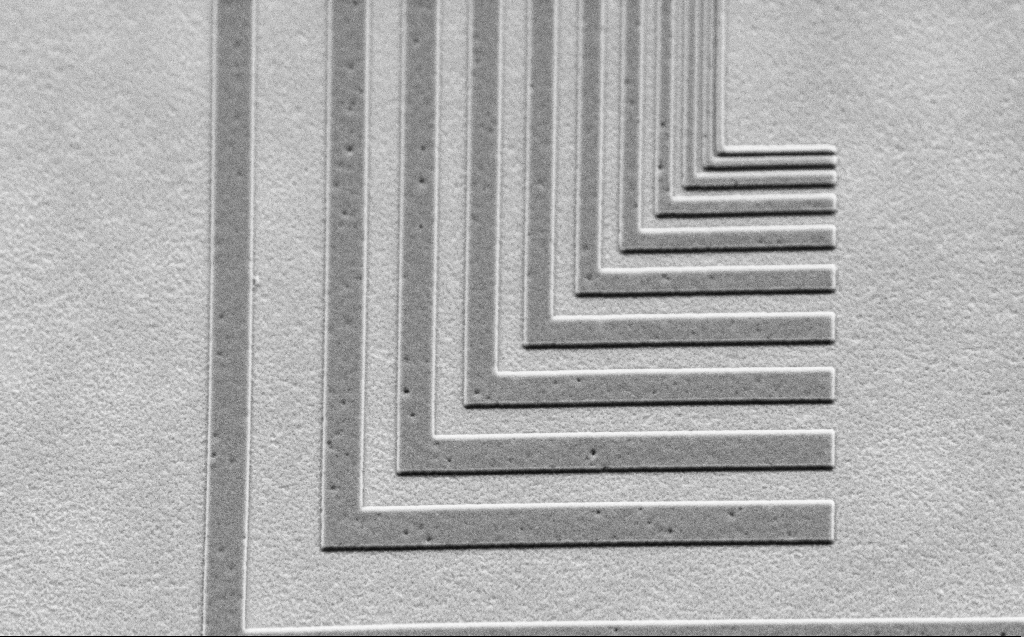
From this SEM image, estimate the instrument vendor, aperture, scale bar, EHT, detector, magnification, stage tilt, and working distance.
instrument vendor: Zeiss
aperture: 30 µm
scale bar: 2000 nm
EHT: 2.5 kV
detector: SE2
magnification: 14.59 K X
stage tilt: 44.1°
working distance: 6 mm